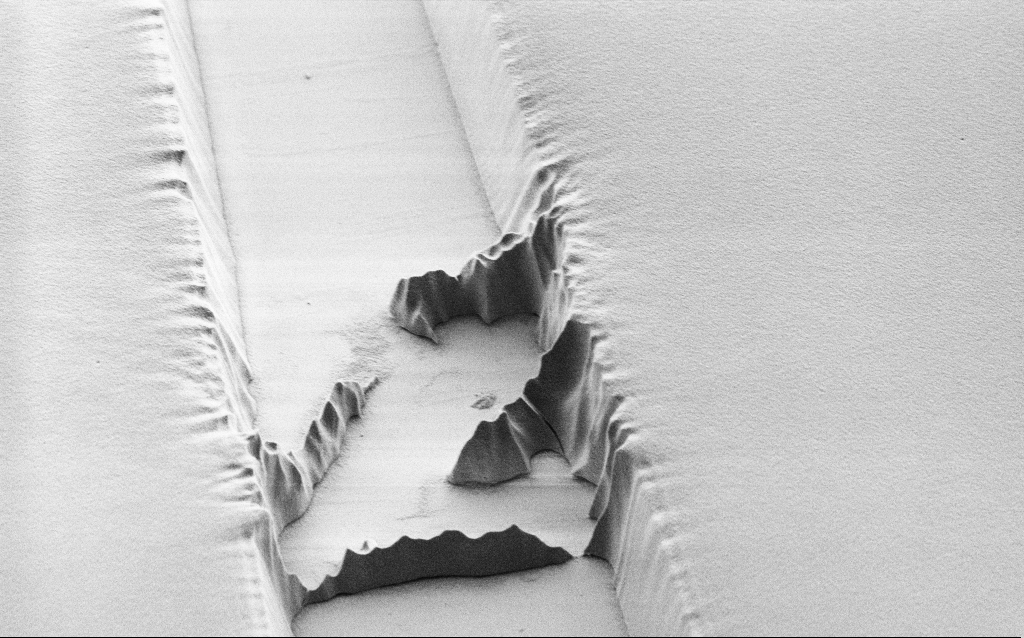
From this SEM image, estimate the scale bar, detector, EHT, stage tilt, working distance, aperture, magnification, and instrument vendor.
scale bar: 2000 nm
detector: SE2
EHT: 1.2 kV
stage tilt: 45°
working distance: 8 mm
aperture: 30 µm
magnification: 8.26 K X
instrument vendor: Zeiss